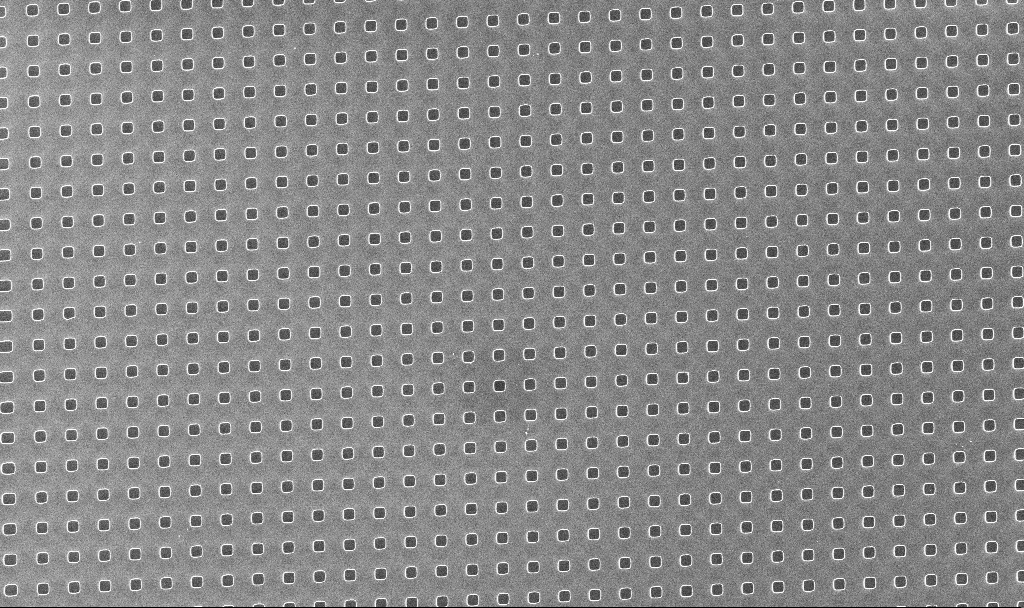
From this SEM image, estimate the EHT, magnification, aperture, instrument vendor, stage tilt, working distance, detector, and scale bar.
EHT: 5 kV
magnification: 0.939 K X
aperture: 30 µm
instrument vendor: Zeiss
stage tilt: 0°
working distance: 5.3 mm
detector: InLens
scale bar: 20000 nm